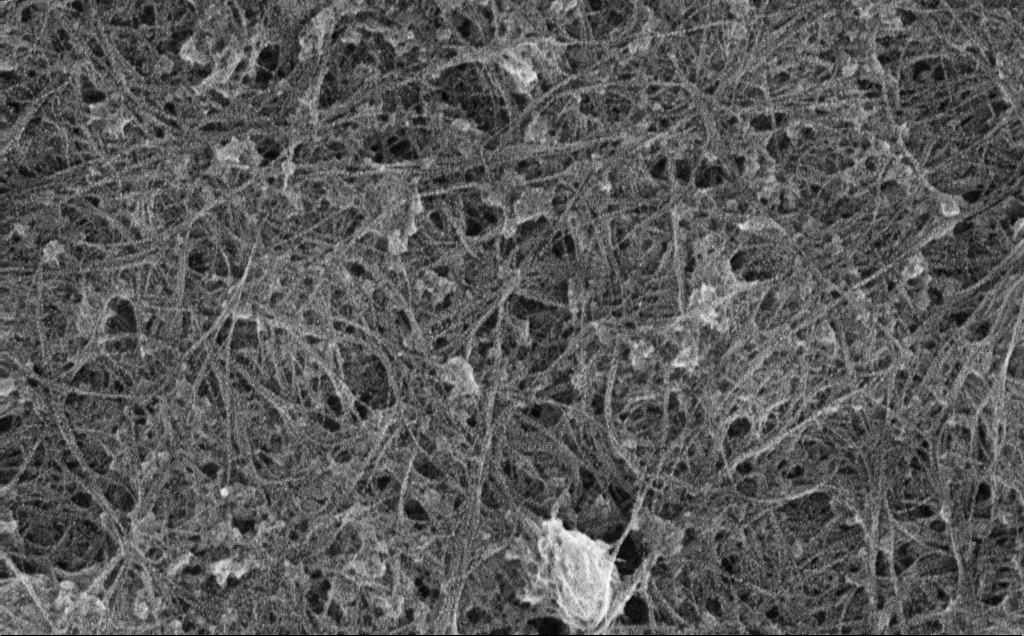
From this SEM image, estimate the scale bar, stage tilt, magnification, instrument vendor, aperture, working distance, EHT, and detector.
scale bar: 1000 nm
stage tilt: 0°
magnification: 40.95 K X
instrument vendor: Zeiss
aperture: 30 µm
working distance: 3 mm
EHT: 10 kV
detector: InLens